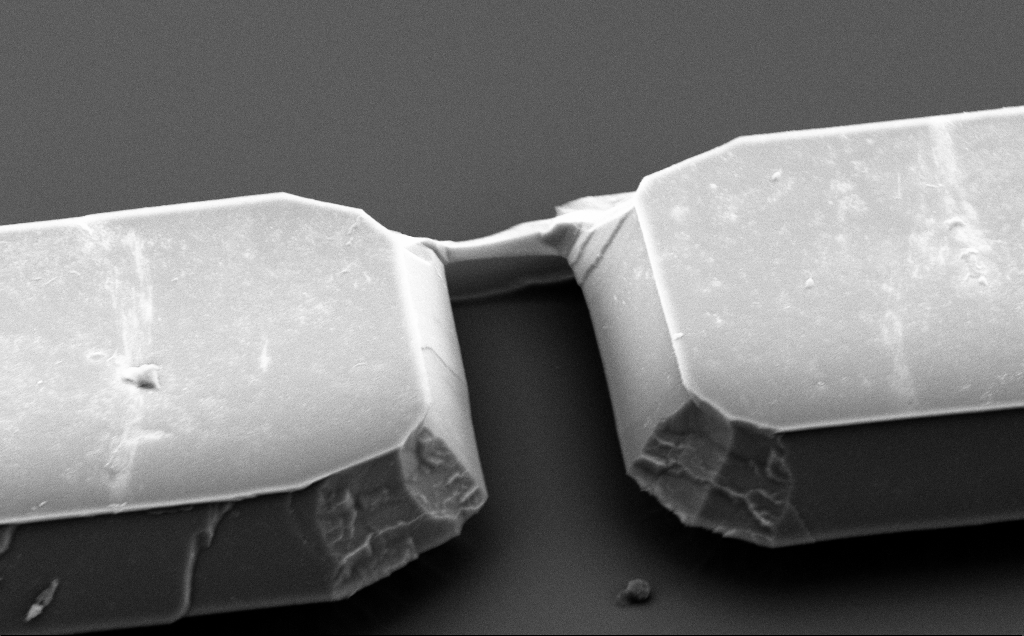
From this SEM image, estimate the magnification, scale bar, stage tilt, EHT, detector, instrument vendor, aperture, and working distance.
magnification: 12.5 K X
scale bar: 2000 nm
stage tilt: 50°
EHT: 5 kV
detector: SE2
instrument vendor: Zeiss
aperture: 30 µm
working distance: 10 mm